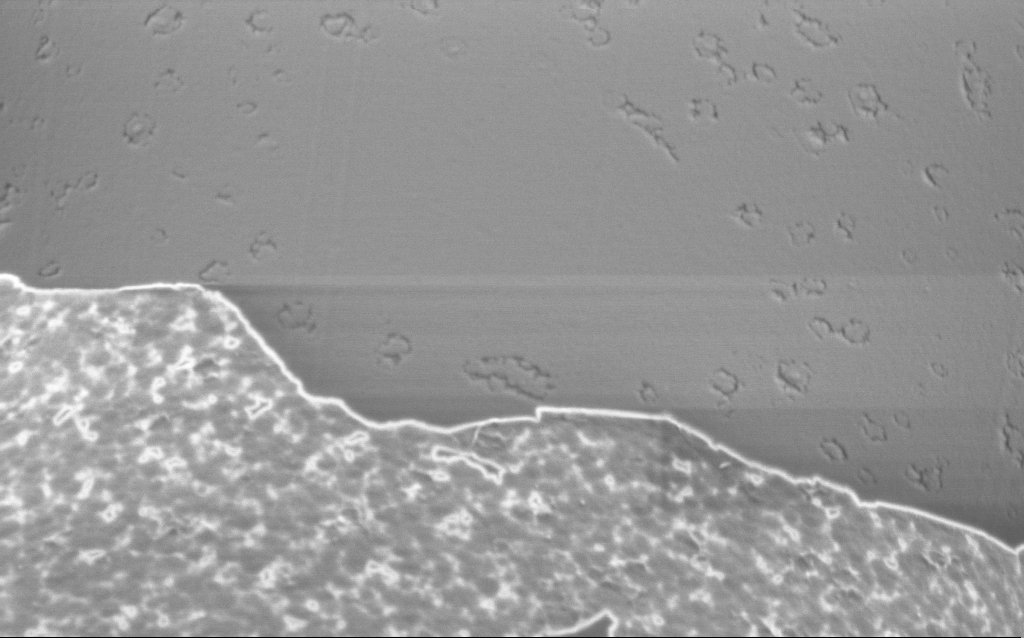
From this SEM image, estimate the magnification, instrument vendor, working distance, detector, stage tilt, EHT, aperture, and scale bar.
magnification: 50 K X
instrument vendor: Zeiss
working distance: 6 mm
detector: InLens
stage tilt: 45°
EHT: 1 kV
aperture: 30 µm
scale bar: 1000 nm